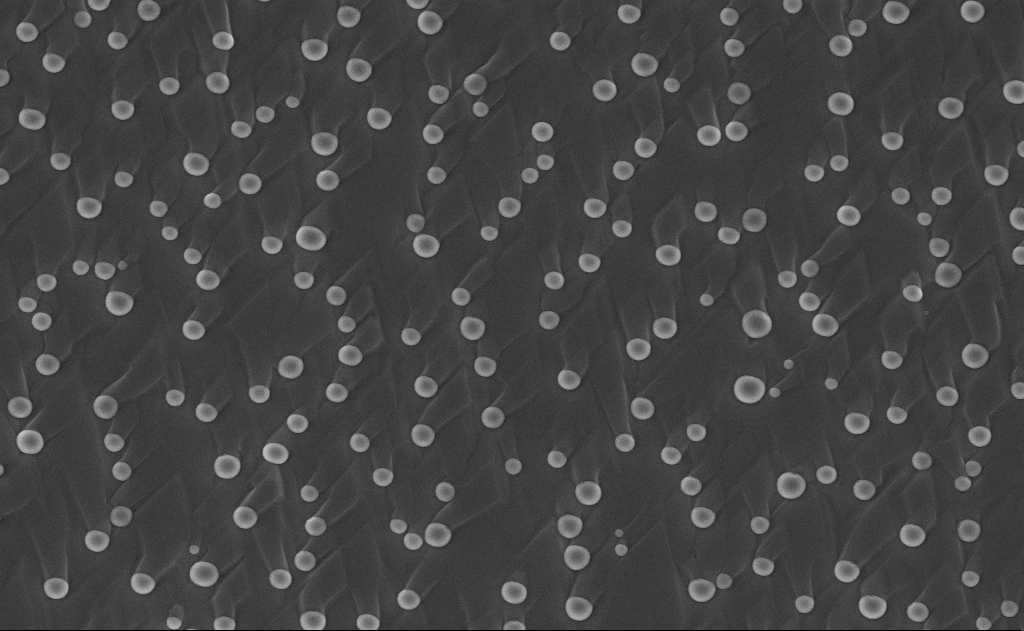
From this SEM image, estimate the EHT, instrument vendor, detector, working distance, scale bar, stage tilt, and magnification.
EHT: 10 kV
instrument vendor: Zeiss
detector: InLens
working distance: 10 mm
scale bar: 2000 nm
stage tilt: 0°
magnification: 10 K X